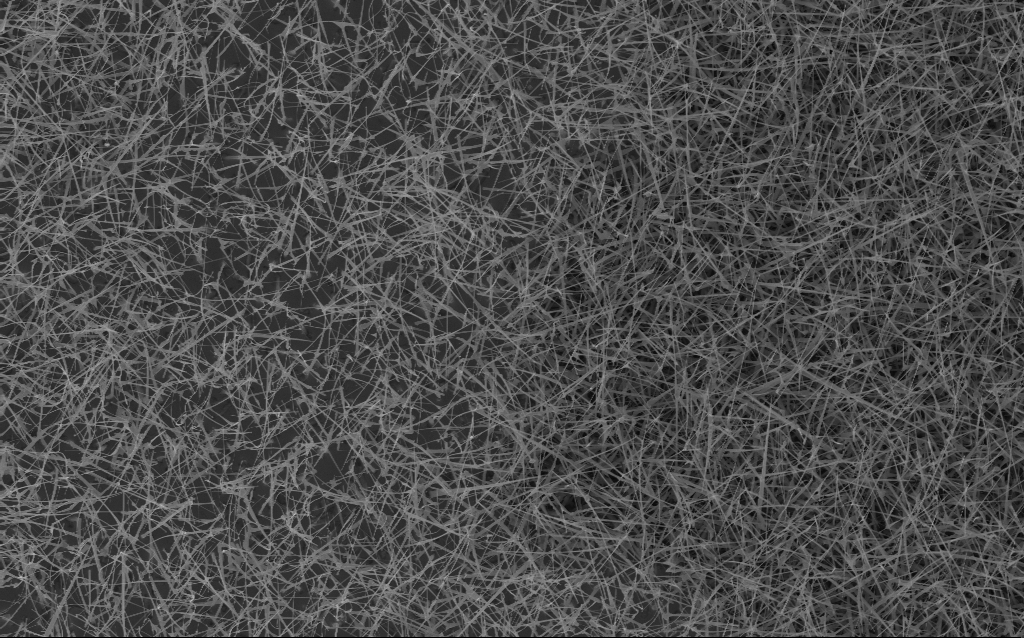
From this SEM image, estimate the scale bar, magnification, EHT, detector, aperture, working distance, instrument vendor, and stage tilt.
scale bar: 10000 nm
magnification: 5 K X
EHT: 10 kV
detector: InLens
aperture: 30 µm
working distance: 6 mm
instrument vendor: Zeiss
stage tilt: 0°